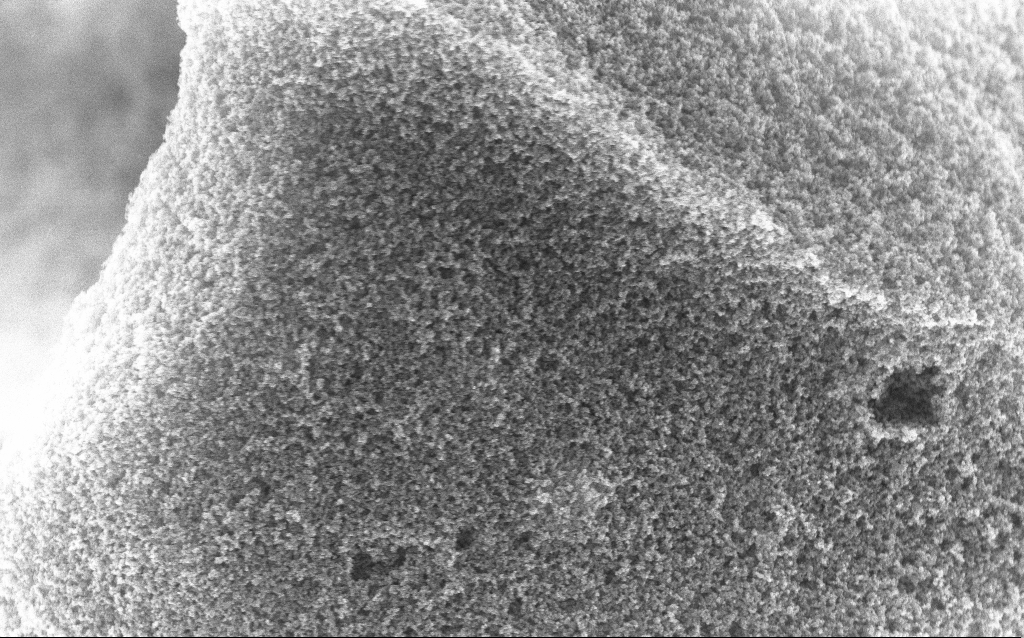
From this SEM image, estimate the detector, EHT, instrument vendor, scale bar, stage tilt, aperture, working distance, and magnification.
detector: InLens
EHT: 10 kV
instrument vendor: Zeiss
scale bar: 1000 nm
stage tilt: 0°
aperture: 30 µm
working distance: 2.7 mm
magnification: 37.88 K X